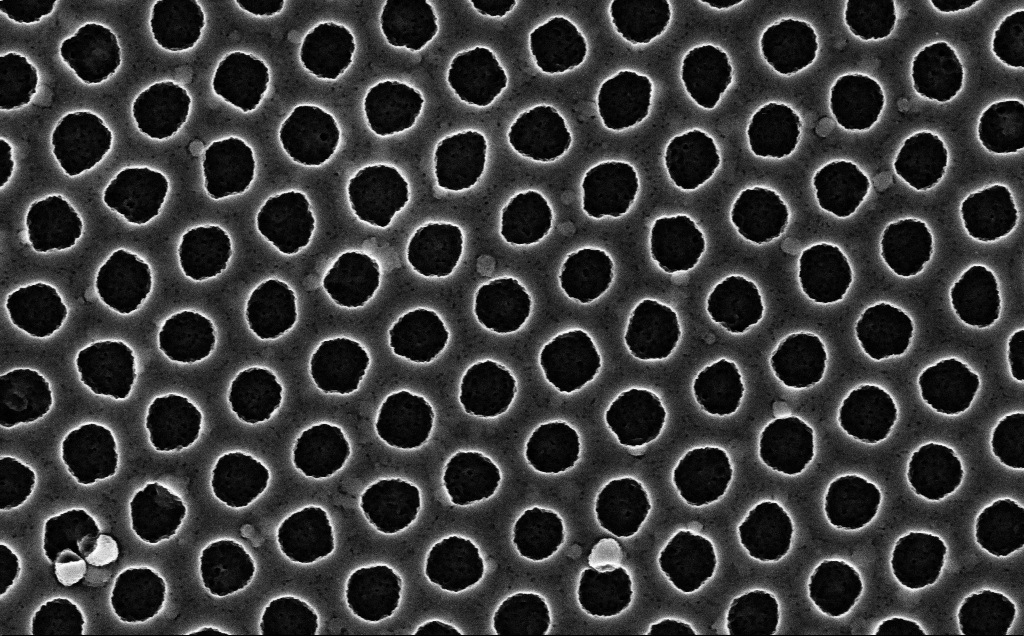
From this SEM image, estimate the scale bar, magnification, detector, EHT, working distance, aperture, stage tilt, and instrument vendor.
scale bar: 200 nm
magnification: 90 K X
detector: InLens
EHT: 3 kV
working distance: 2.5 mm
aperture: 30 µm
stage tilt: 0°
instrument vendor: Zeiss